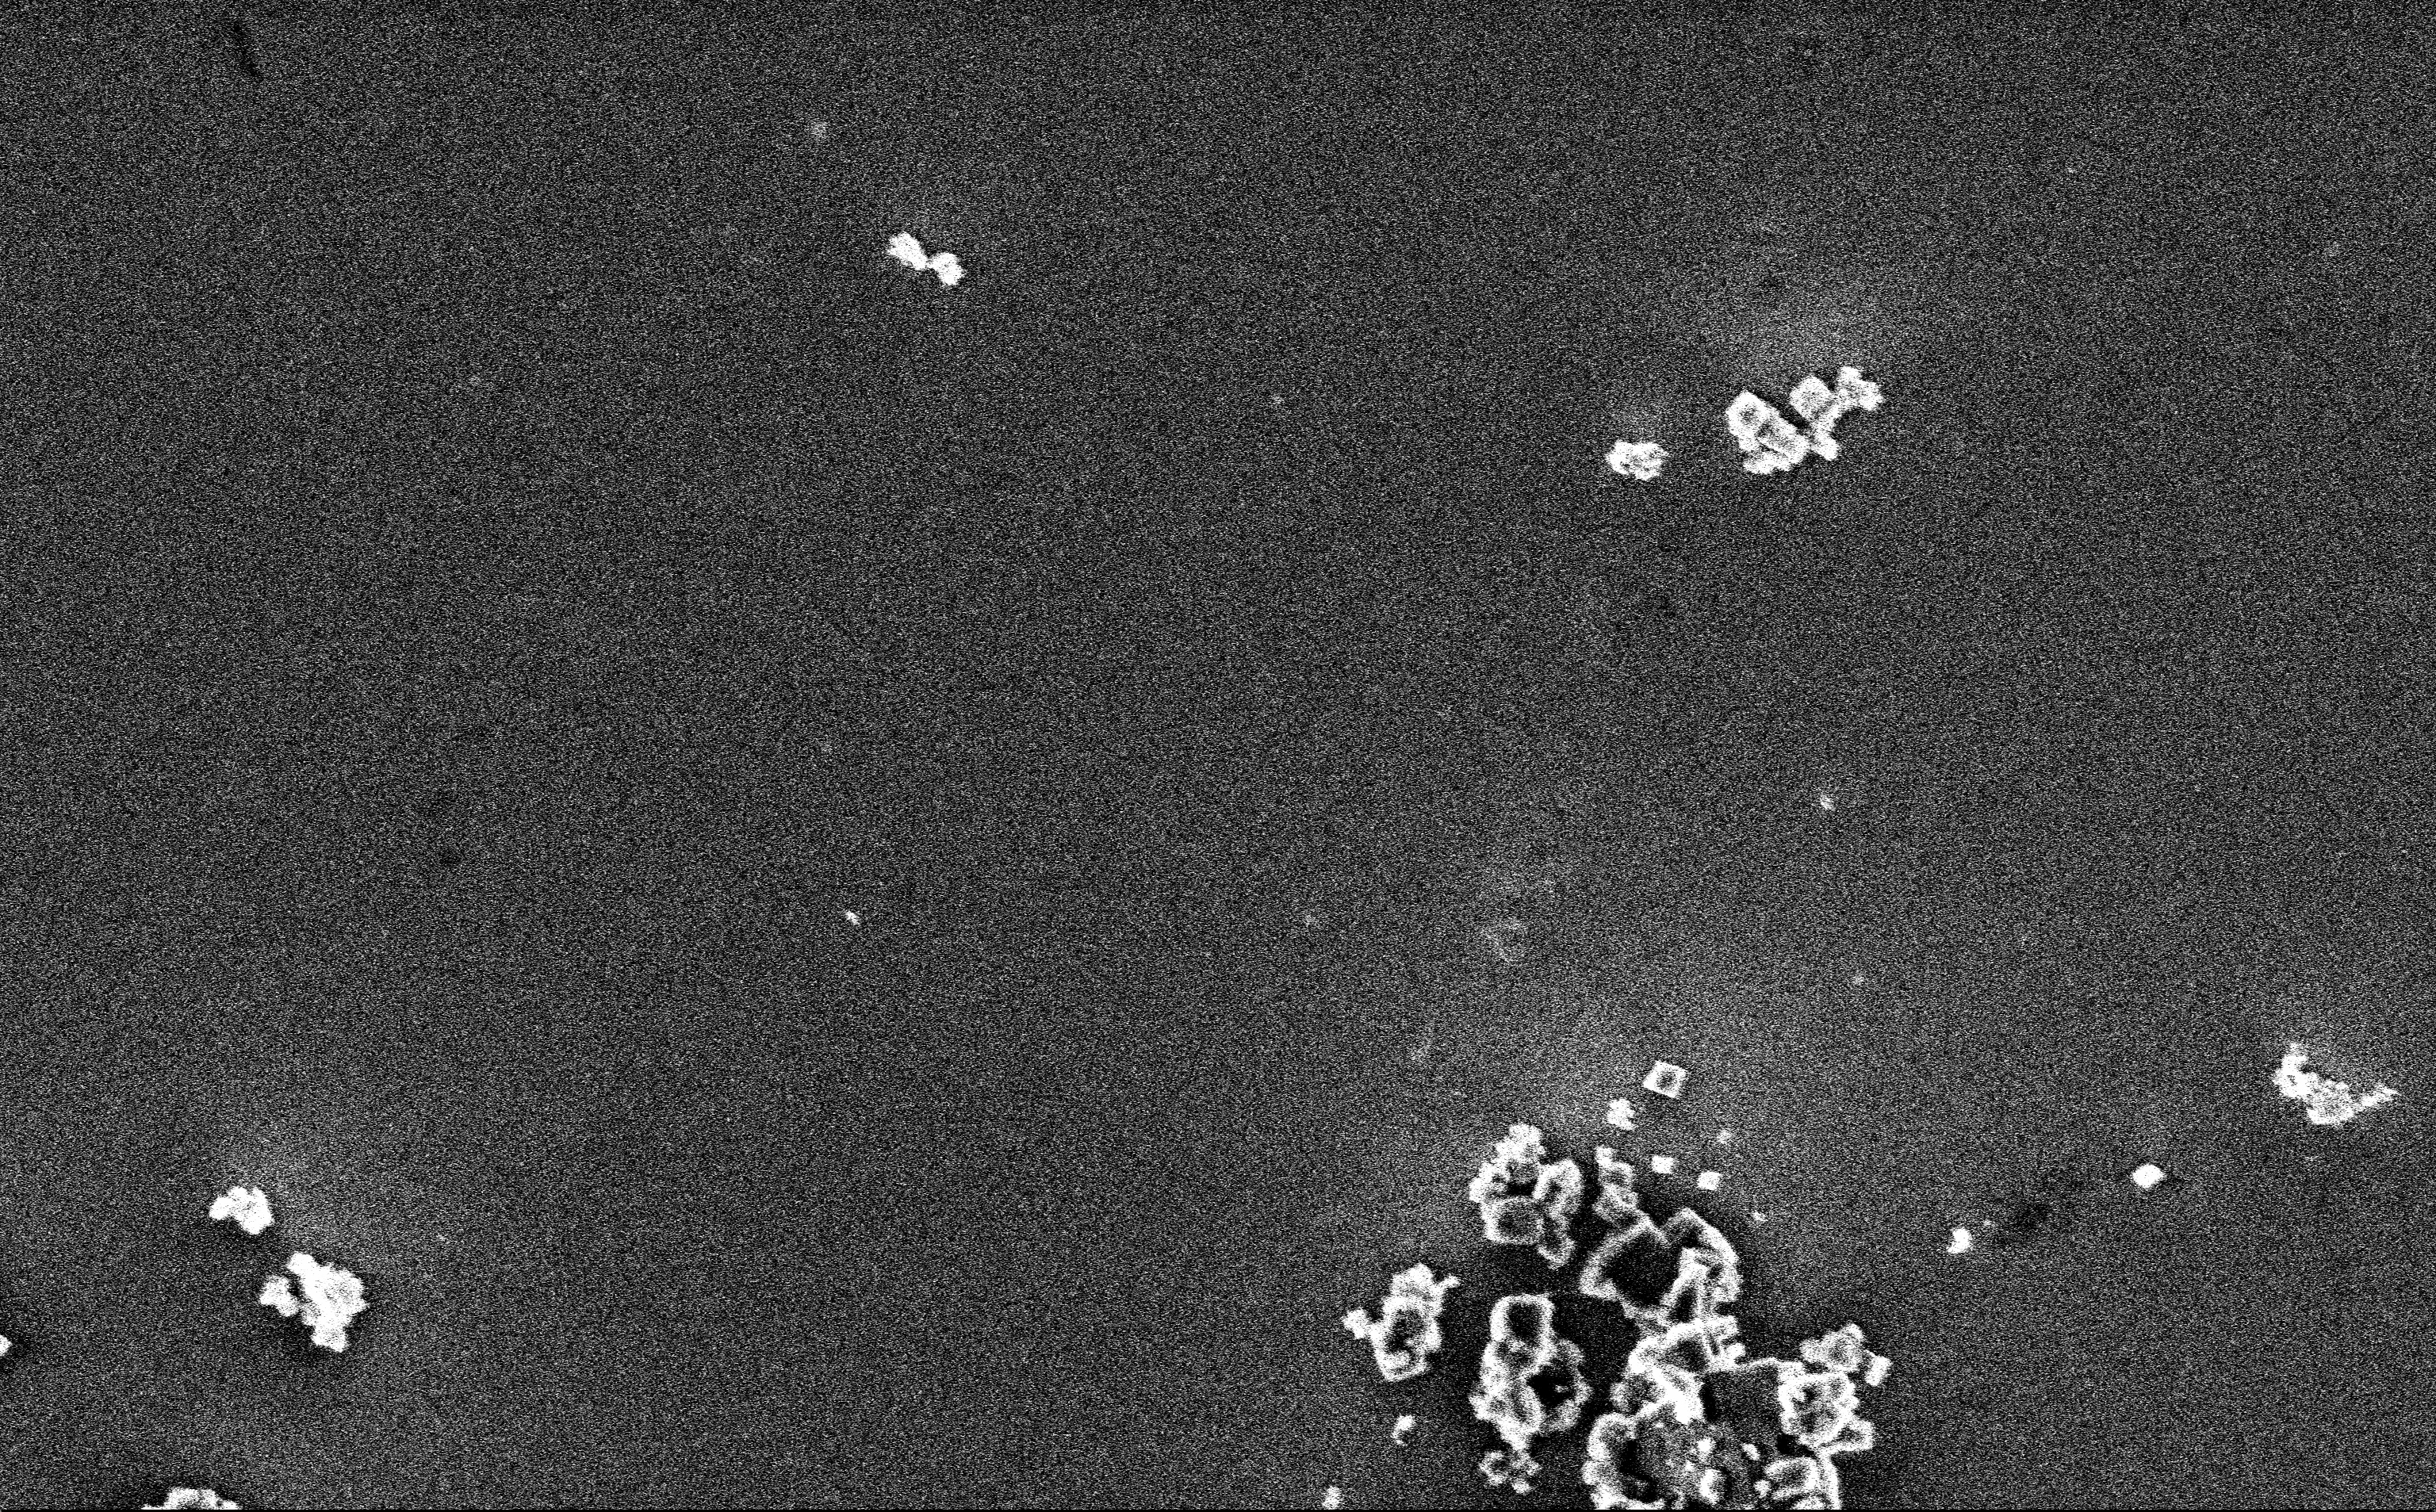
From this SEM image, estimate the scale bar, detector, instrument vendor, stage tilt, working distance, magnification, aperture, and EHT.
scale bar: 2000 nm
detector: InLens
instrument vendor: Zeiss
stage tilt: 0°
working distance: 3 mm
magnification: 19.01 K X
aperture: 30 µm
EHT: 3 kV